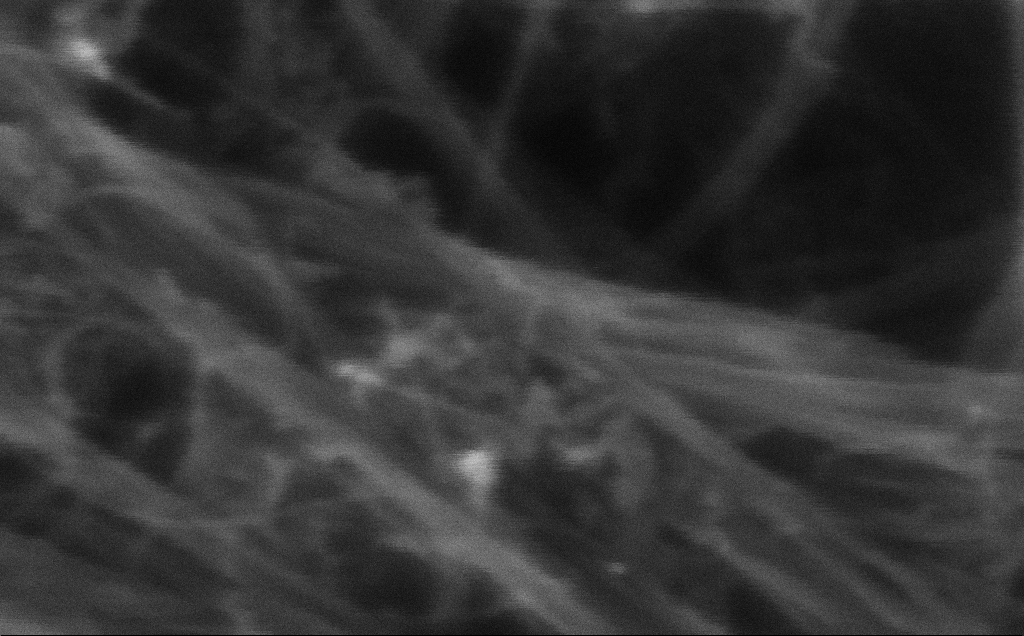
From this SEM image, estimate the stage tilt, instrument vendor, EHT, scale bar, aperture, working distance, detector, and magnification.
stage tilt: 0°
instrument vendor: Zeiss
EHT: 10 kV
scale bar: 20 nm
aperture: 30 µm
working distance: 3 mm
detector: InLens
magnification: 962.68 K X